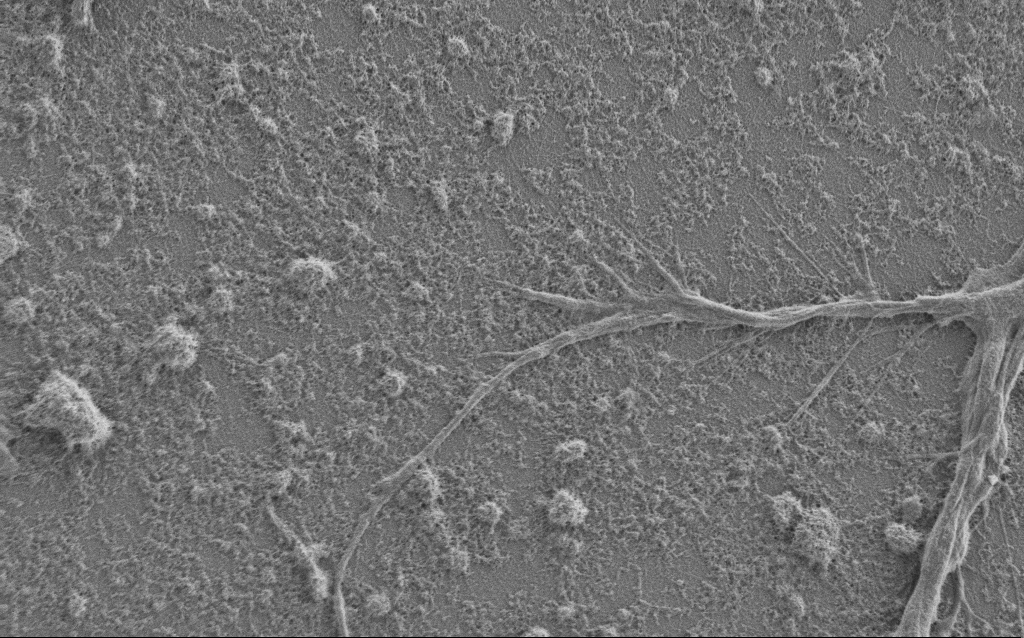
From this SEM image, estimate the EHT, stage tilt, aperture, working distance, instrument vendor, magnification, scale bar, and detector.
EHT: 1 kV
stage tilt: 0°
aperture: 30 µm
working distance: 6 mm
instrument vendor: Zeiss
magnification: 7.5 K X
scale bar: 2000 nm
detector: SE2